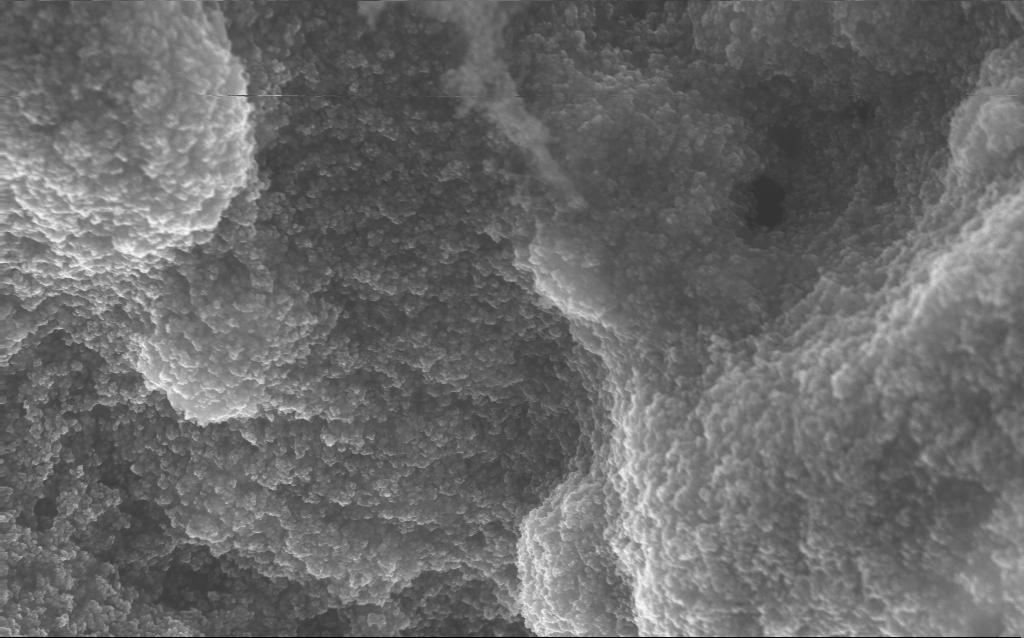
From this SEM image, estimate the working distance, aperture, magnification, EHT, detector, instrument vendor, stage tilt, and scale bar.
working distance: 2.8 mm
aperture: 30 µm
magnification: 37.88 K X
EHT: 10 kV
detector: InLens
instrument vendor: Zeiss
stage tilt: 0°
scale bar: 1000 nm